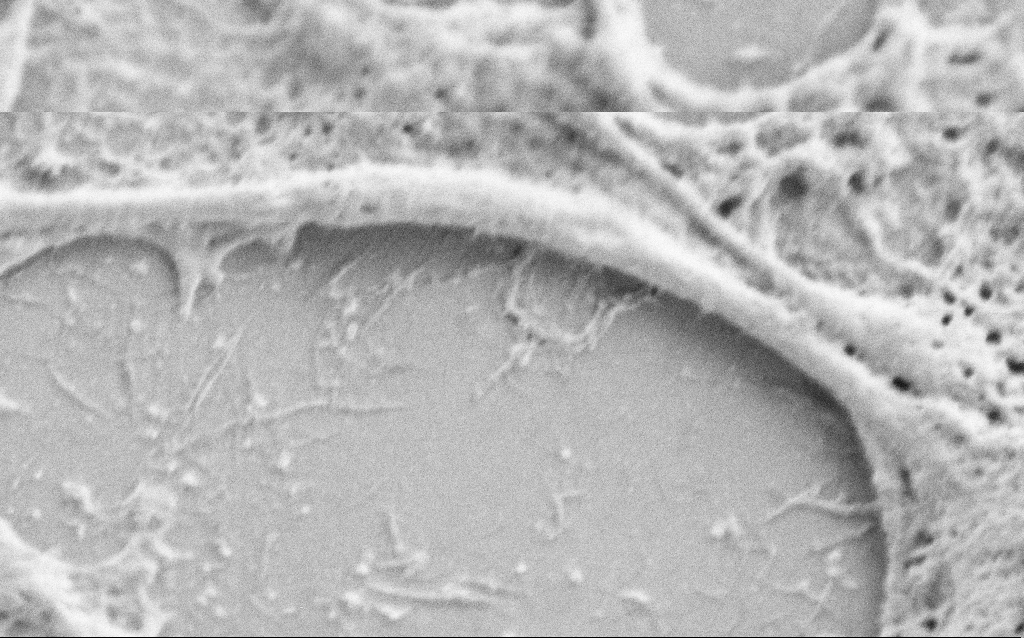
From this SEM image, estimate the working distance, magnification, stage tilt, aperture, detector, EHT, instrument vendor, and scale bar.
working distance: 5.8 mm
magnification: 40 K X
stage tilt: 0°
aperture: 30 µm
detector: SE2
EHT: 0.8 kV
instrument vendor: Zeiss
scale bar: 1000 nm